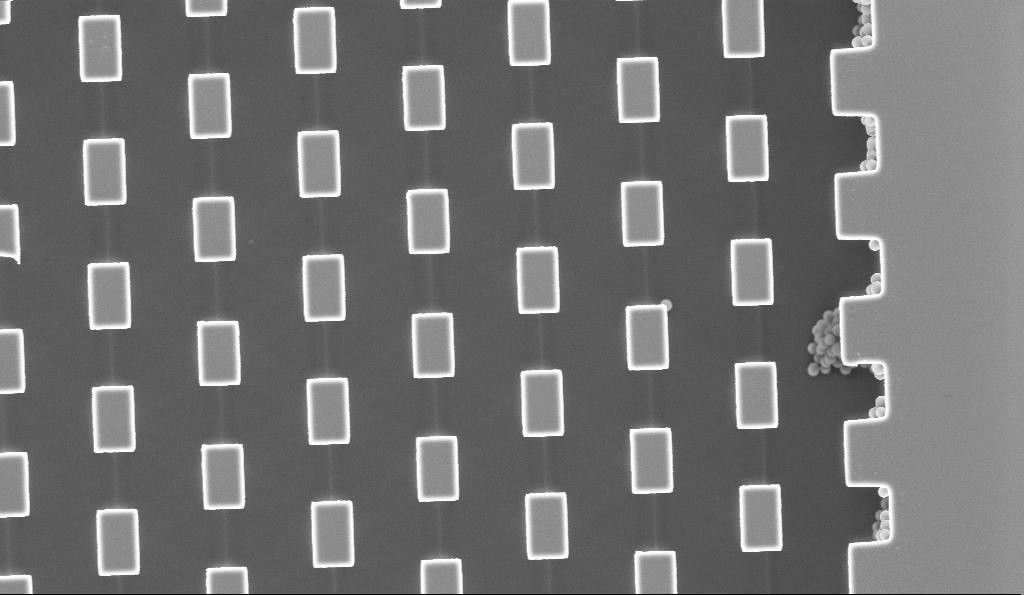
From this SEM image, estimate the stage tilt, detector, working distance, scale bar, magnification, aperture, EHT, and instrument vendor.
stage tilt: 0°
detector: InLens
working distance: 3.1 mm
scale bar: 2000 nm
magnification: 7.57 K X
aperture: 30 µm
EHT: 5 kV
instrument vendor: Zeiss